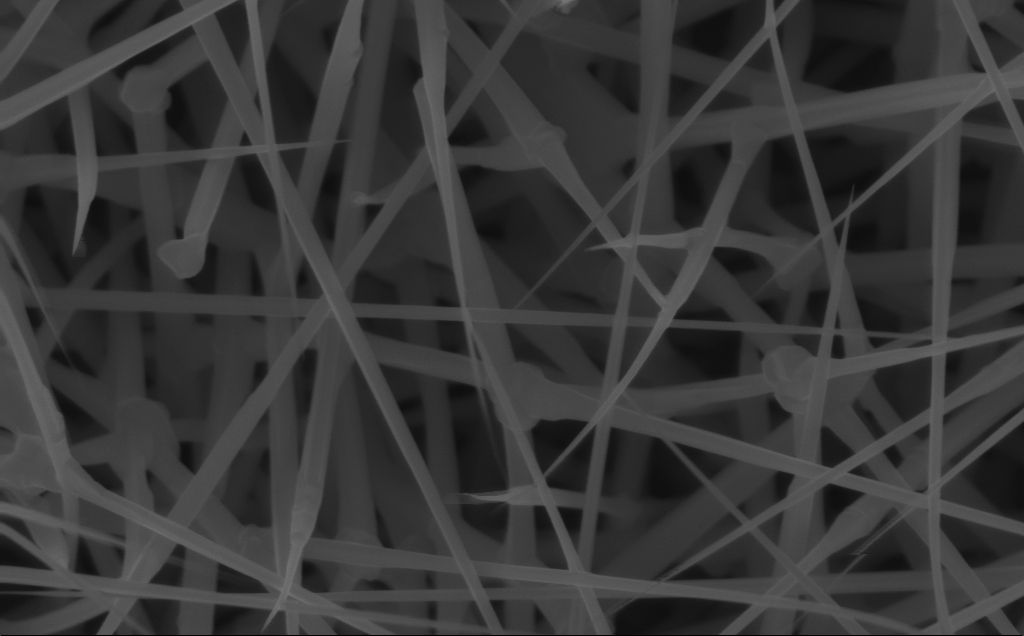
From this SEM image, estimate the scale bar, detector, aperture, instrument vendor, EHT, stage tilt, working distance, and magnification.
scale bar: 200 nm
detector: InLens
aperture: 30 µm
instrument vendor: Zeiss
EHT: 10 kV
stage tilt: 0°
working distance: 6 mm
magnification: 80 K X